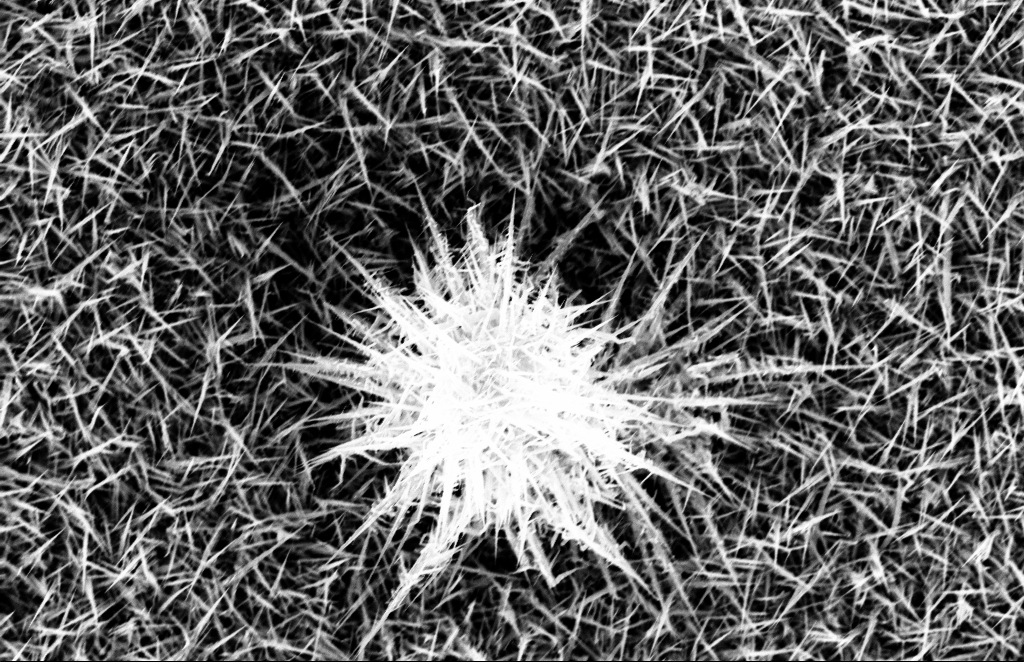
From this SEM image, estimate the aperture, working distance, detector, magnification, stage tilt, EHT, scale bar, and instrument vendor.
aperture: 30 µm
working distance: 10 mm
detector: InLens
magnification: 22.35 K X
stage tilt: -1.1°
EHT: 10 kV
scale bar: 2000 nm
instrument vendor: Zeiss